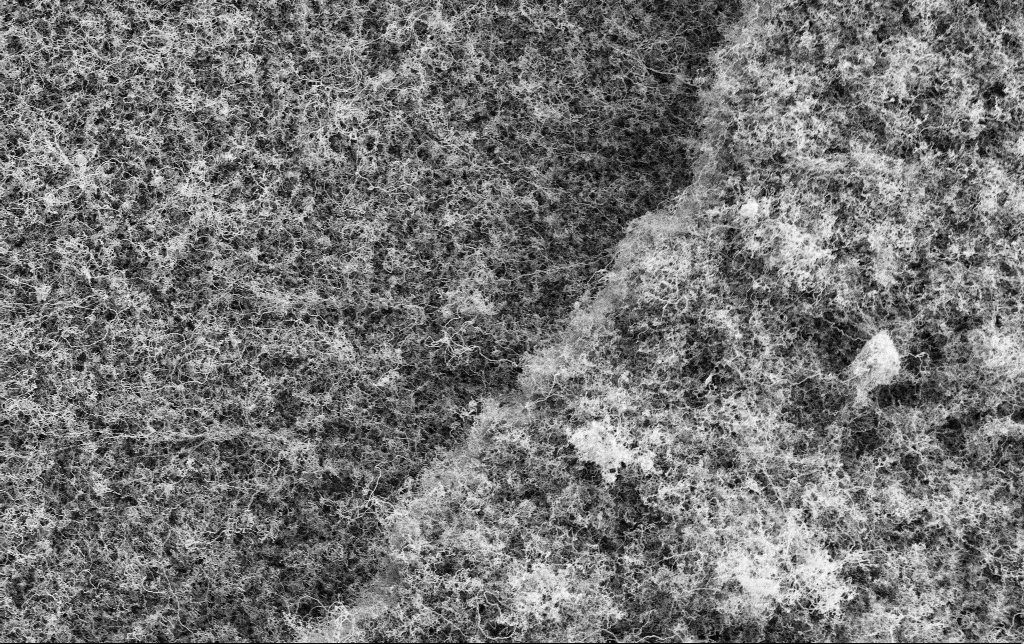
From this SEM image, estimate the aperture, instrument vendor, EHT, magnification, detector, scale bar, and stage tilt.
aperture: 30 µm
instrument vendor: Zeiss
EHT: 2 kV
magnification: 5 K X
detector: SE2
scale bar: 10000 nm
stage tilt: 0°